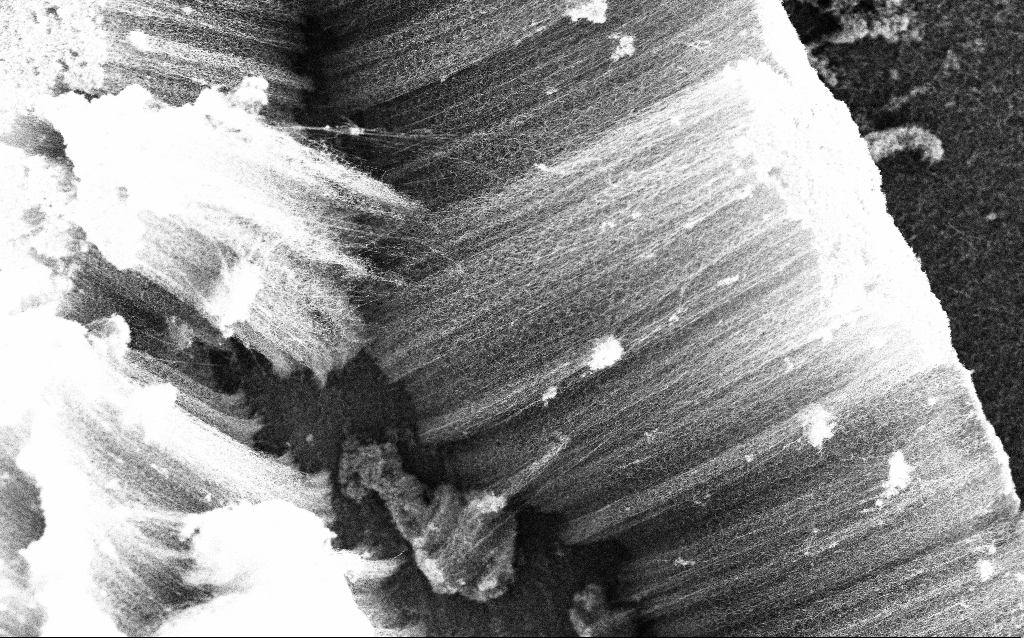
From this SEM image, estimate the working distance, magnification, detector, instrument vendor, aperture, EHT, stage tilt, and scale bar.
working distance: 2.3 mm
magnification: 3.19 K X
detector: InLens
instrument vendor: Zeiss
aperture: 30 µm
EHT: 5 kV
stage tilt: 0°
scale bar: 10000 nm